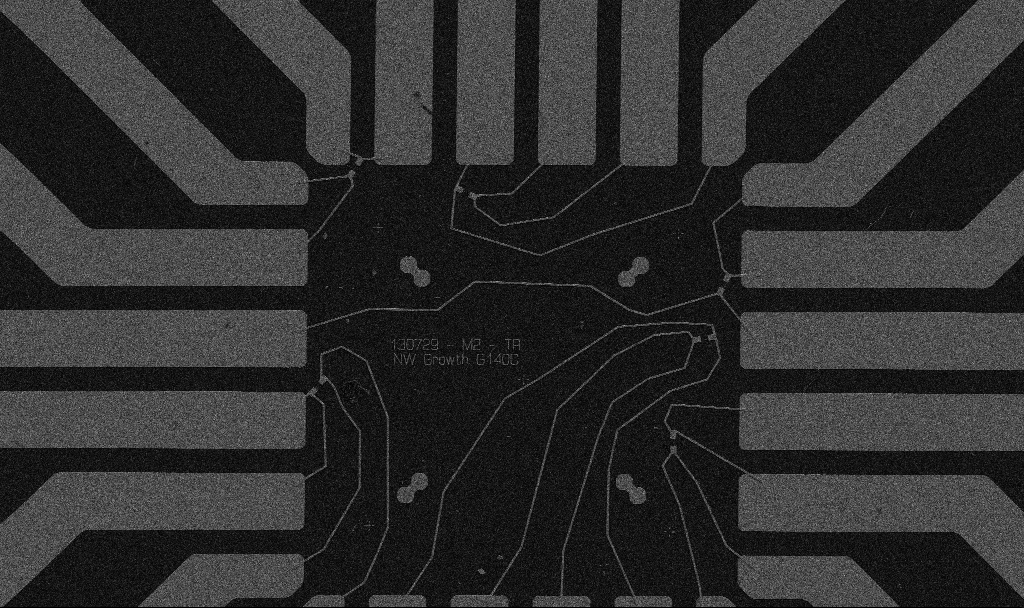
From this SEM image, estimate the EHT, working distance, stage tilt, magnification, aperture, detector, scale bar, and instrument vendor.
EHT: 5 kV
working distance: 10.7 mm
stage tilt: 0°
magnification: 1 K X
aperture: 30 µm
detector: SE2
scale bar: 20000 nm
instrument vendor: Zeiss